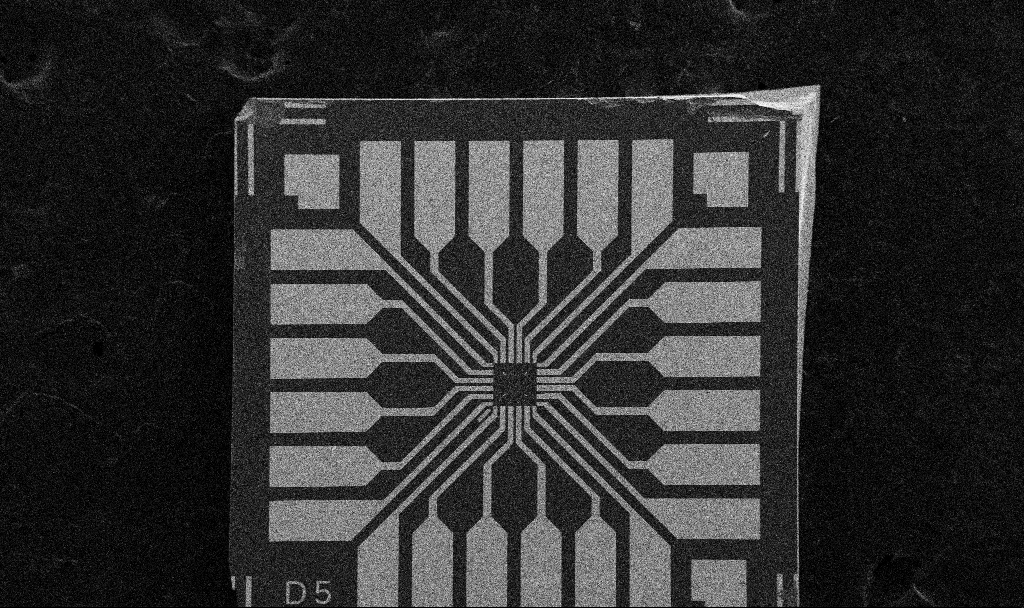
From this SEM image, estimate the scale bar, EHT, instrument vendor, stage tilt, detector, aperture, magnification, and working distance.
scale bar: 200000 nm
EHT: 5 kV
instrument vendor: Zeiss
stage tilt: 0°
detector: SE2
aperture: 30 µm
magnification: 0.1 K X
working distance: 10.7 mm